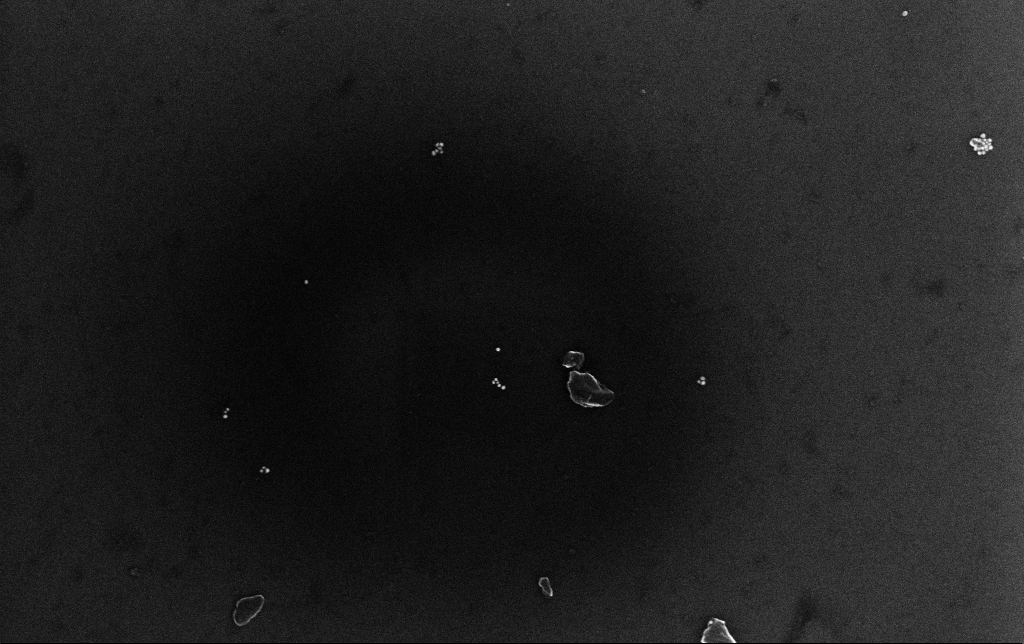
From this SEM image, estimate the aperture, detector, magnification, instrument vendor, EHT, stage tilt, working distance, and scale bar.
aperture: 30 µm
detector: InLens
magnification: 100 K X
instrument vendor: Zeiss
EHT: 10 kV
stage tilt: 0°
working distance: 3.4 mm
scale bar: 200 nm